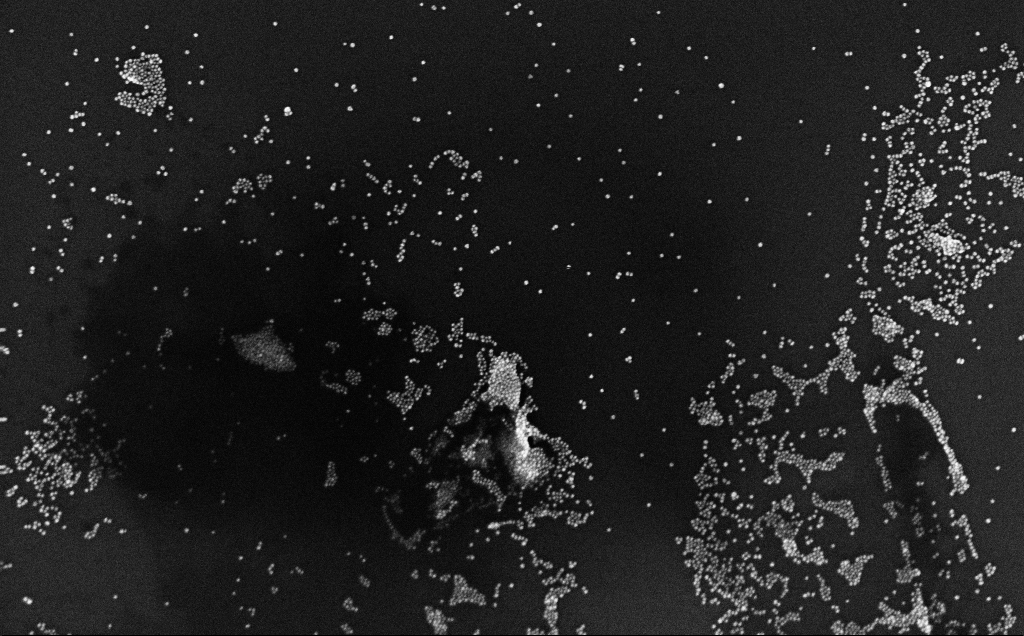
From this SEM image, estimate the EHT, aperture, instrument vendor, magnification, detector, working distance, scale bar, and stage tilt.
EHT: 10 kV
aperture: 30 µm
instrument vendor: Zeiss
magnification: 100 K X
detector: InLens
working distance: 3.2 mm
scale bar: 200 nm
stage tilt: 0°